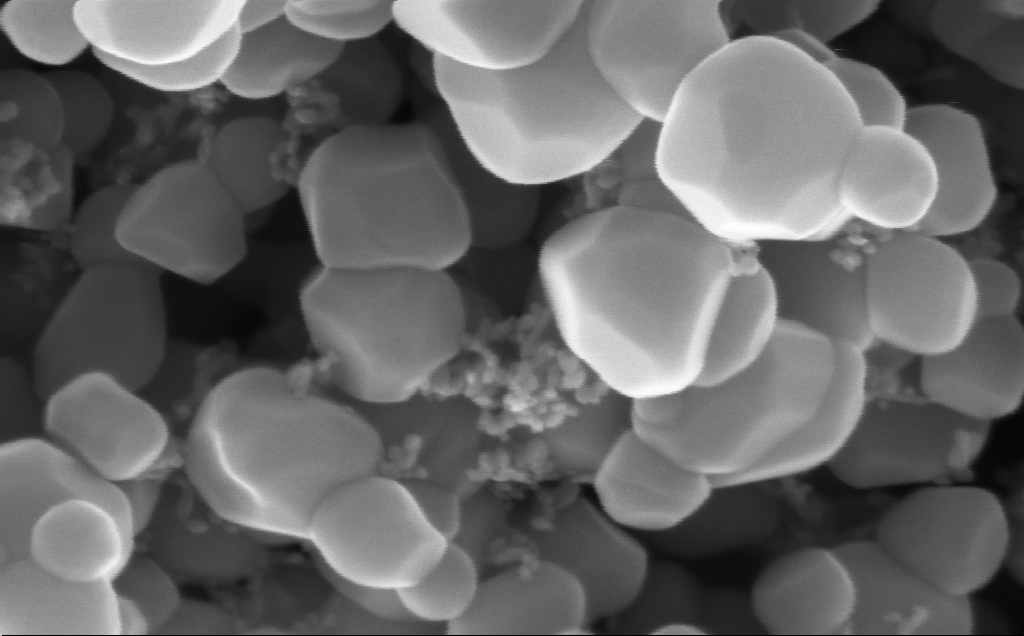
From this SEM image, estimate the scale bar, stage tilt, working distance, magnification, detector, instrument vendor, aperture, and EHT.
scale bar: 100 nm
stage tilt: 0°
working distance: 2.3 mm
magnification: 416 K X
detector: InLens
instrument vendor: Zeiss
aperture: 30 µm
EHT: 5 kV